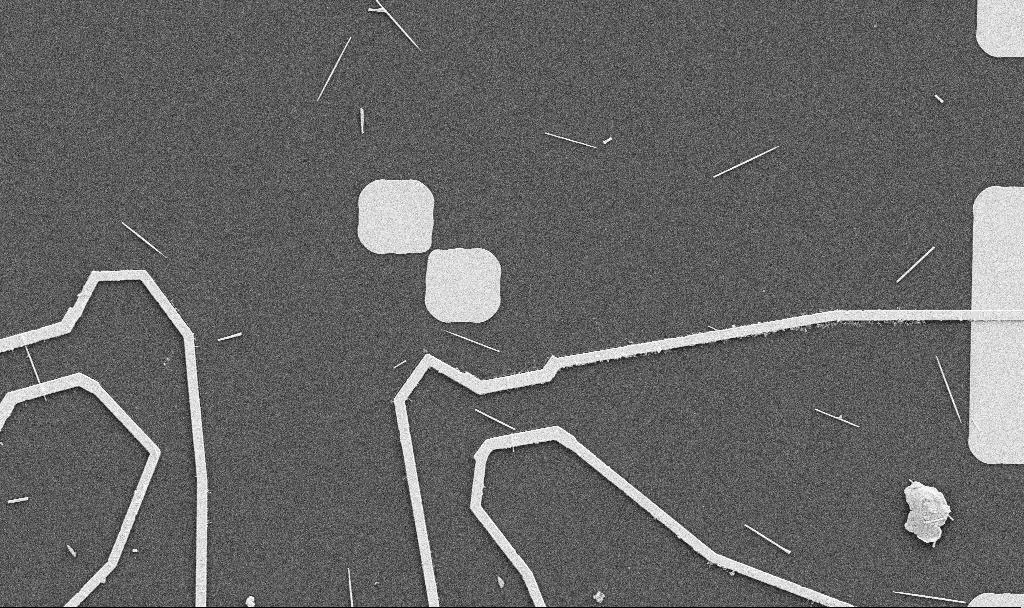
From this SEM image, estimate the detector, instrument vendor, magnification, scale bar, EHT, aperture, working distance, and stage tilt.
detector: SE2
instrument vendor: Zeiss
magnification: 5 K X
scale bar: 10000 nm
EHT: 5 kV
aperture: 30 µm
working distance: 10.7 mm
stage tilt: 0°